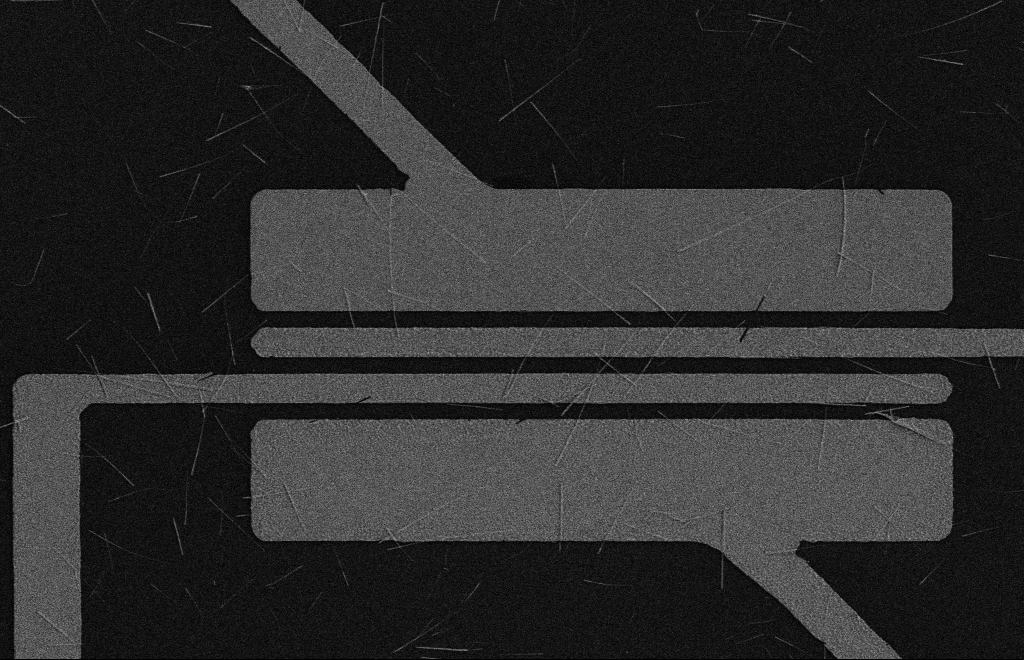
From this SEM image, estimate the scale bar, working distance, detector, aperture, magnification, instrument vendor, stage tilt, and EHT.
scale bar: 10000 nm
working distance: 16 mm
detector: SE2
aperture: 10 µm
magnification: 4.26 K X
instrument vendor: Zeiss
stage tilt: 0°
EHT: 5 kV